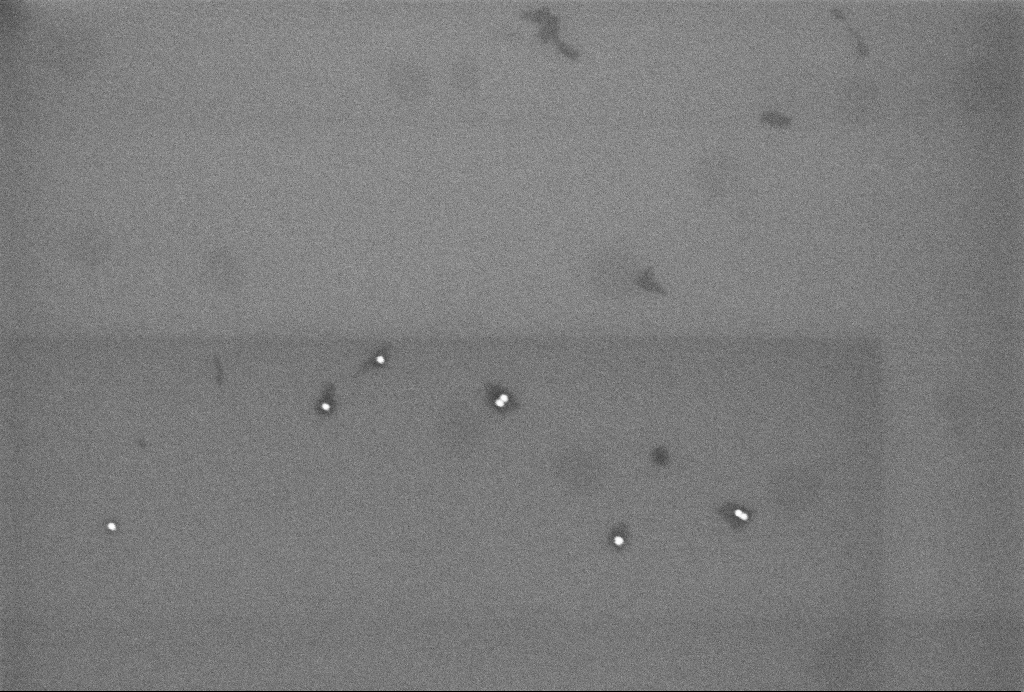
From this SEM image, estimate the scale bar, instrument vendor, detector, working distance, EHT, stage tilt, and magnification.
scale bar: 200 nm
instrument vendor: Zeiss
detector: InLens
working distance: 3.2 mm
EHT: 3 kV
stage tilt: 0°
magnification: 99.96 K X